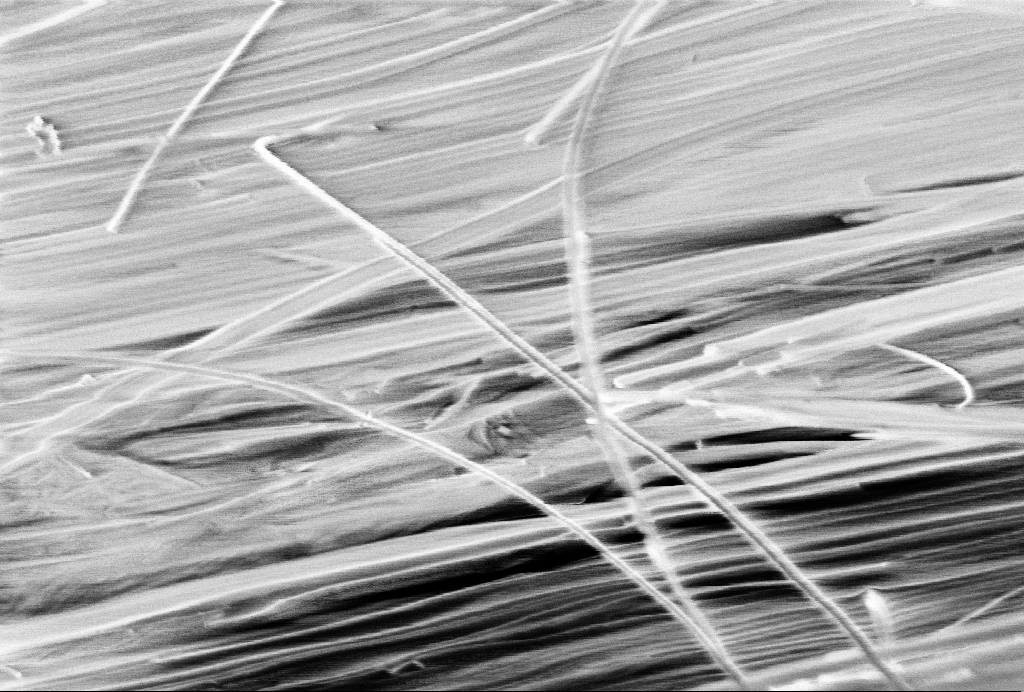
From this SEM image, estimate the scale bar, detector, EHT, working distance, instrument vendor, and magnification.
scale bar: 200 nm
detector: InLens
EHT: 1 kV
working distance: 6.8 mm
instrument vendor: Zeiss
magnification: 64.27 K X